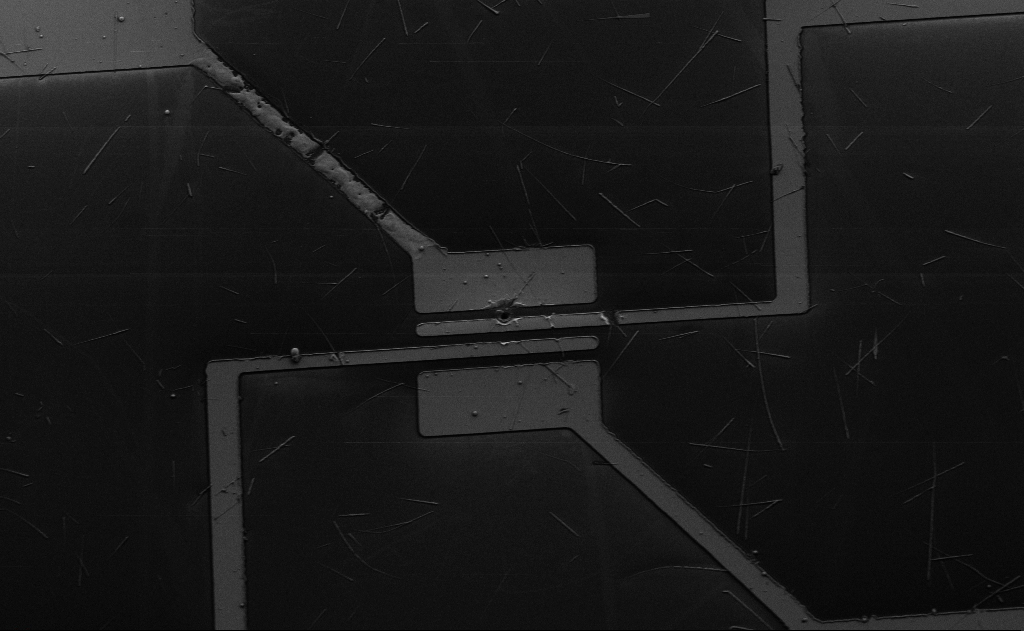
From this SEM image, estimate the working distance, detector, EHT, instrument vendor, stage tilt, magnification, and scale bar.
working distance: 15 mm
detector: SE2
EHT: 5 kV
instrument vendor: Zeiss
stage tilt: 0°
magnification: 2.29 K X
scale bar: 20000 nm